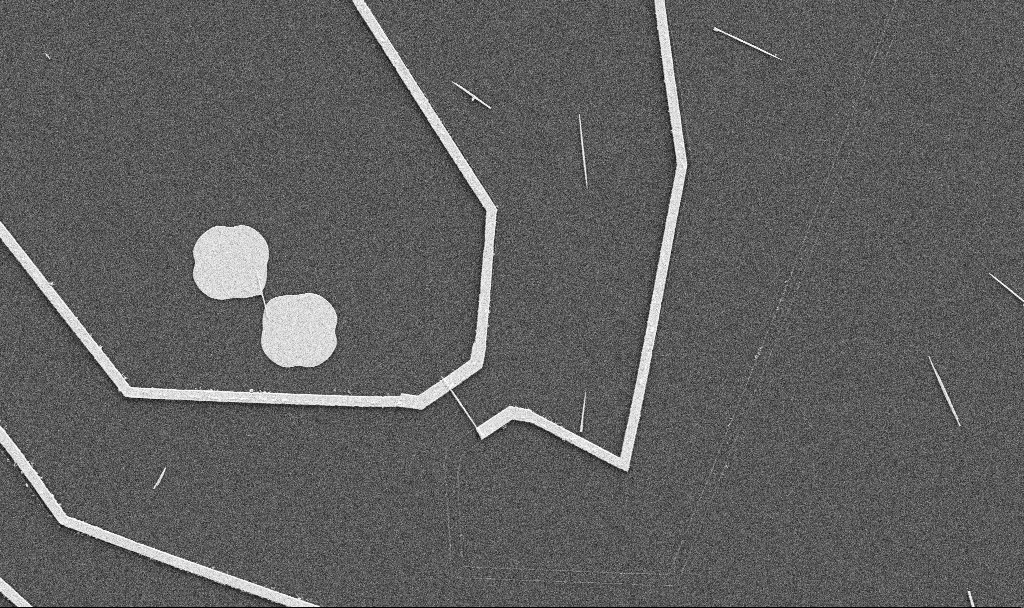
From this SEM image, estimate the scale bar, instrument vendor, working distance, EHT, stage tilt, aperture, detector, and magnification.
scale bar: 10000 nm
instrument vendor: Zeiss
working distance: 10.7 mm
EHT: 5 kV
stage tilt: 0°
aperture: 30 µm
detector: SE2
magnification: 5 K X